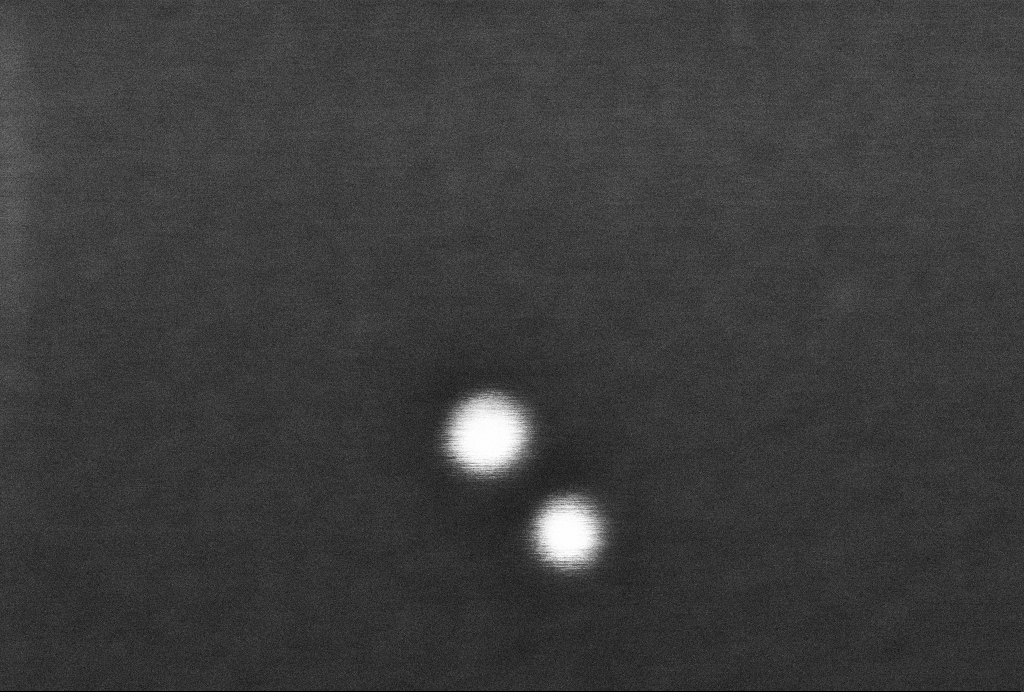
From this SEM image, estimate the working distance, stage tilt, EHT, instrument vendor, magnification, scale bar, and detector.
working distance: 3.3 mm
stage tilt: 0°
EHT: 2 kV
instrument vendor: Zeiss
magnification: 884.88 K X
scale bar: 20 nm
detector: InLens